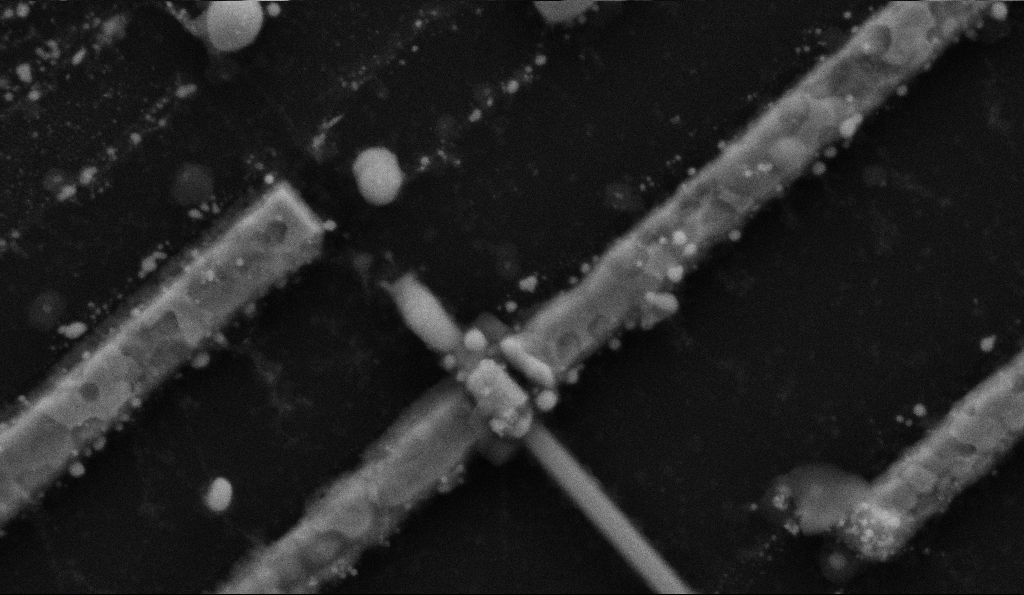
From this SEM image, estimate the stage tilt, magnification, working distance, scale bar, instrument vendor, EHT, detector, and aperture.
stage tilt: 0°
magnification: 100 K X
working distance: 8.5 mm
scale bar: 200 nm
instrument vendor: Zeiss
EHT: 5 kV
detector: SE2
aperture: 30 µm